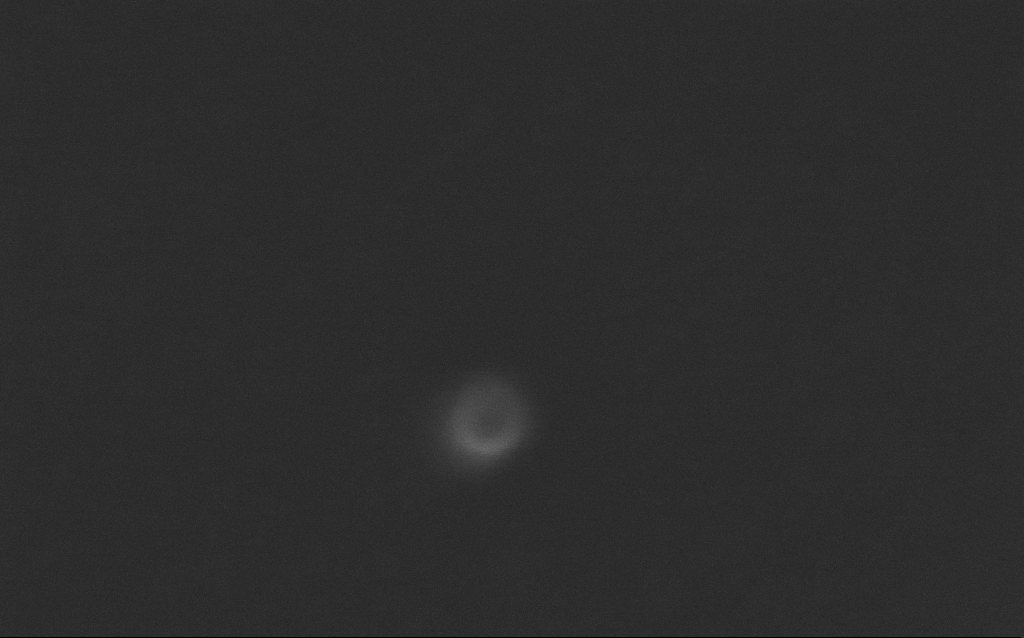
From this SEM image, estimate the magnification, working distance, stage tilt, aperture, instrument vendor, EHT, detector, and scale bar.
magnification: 942.72 K X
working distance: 4 mm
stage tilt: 0°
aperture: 30 µm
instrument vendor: Zeiss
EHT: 10 kV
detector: InLens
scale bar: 20 nm